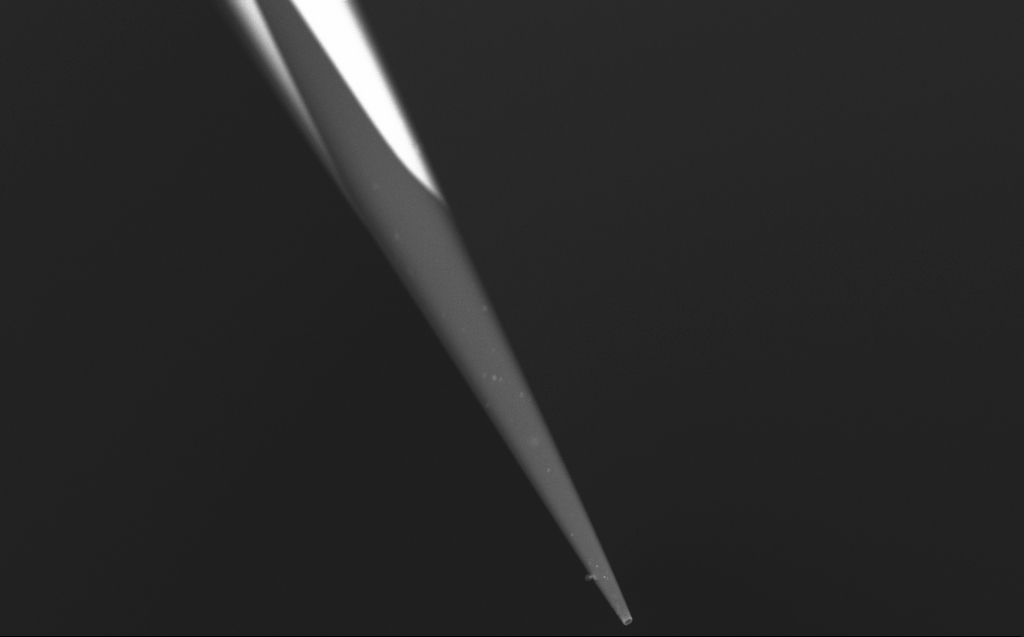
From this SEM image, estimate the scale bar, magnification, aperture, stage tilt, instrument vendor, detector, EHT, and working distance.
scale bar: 10000 nm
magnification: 4.61 K X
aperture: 30 µm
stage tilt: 45°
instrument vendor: Zeiss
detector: InLens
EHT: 5 kV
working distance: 4 mm